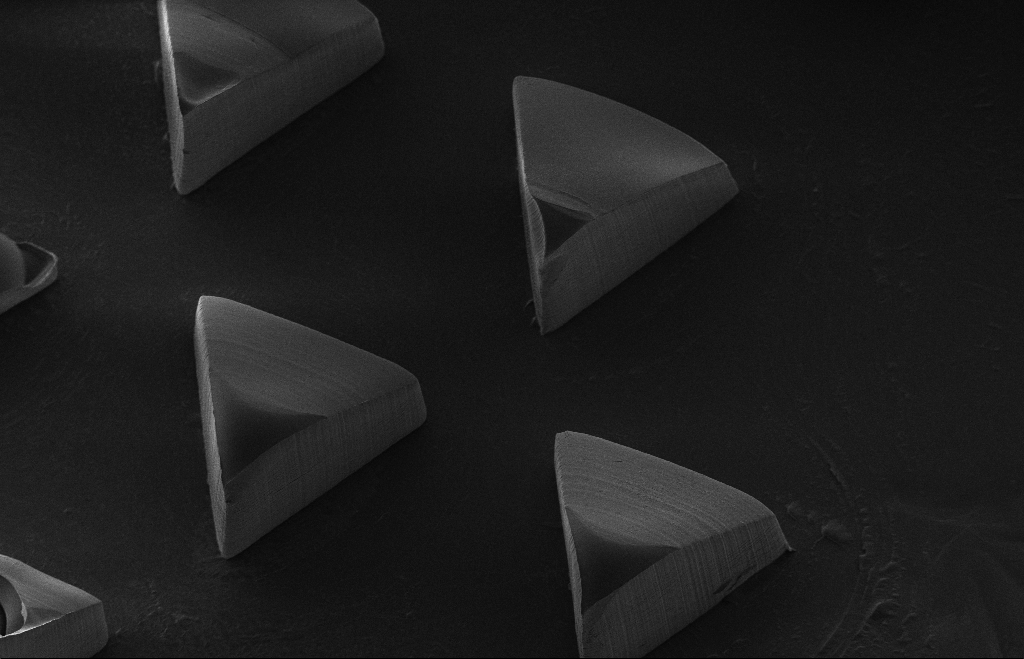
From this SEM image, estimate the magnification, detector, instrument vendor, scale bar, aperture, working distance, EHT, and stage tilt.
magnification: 0.297 K X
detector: SE2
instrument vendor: Zeiss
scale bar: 100000 nm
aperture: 30 µm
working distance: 14 mm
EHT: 5 kV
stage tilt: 17.3°